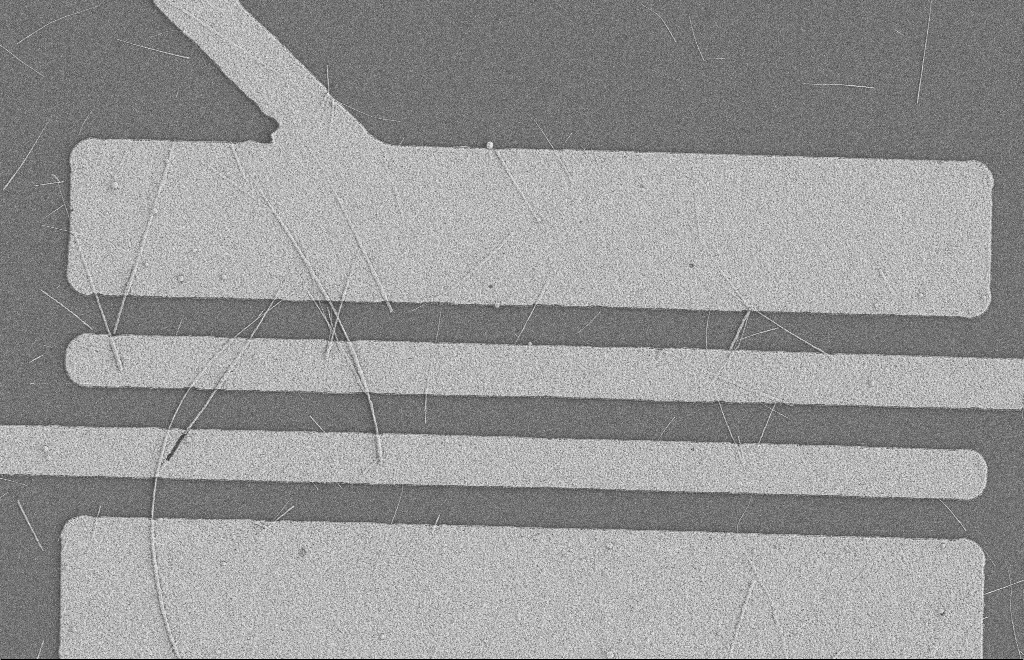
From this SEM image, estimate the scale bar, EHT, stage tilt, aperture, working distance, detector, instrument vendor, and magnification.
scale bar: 2000 nm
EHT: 2 kV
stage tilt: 0°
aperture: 20 µm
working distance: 8 mm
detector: SE2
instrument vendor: Zeiss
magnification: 5.56 K X